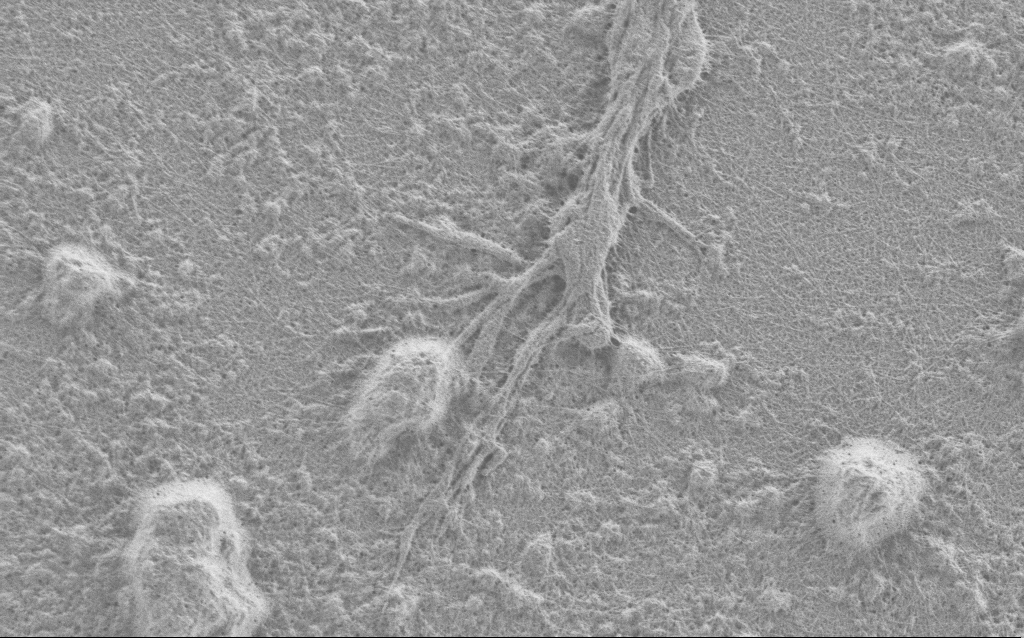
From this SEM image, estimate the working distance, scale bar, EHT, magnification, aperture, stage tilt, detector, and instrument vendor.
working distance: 4 mm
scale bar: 2000 nm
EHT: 0.9 kV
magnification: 10 K X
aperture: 30 µm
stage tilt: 0°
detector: SE2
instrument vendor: Zeiss